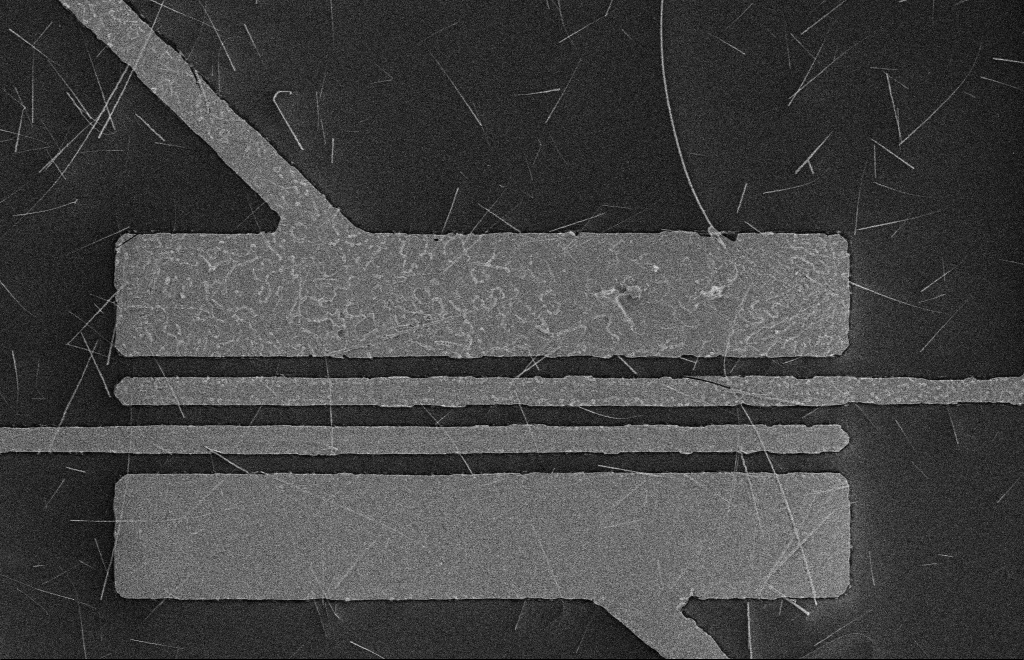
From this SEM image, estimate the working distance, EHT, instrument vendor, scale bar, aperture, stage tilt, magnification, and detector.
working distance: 16 mm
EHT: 5 kV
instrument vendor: Zeiss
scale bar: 10000 nm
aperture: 10 µm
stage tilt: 0°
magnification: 4.47 K X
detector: SE2